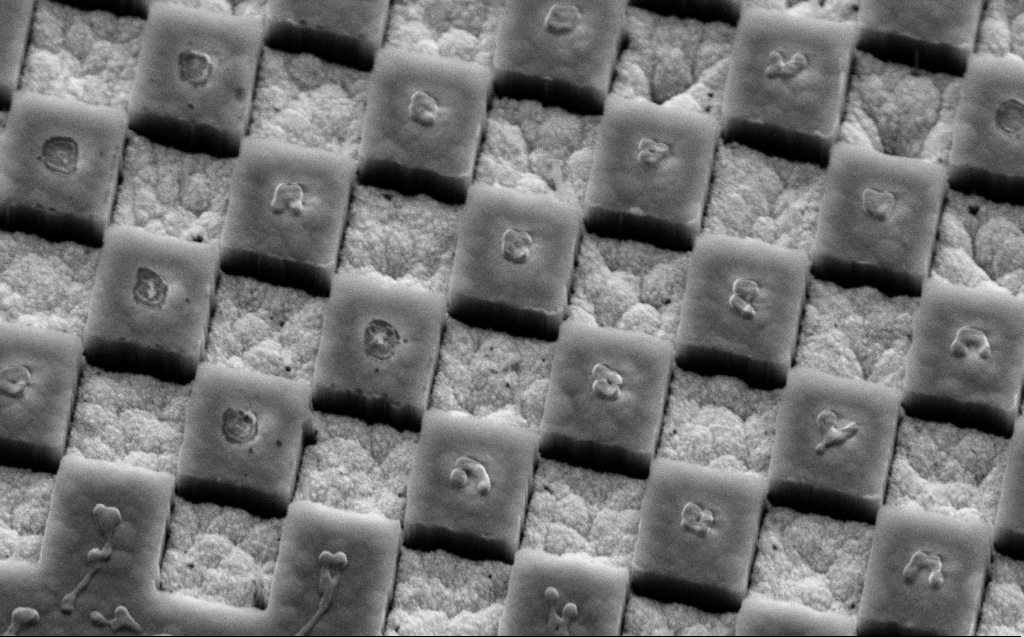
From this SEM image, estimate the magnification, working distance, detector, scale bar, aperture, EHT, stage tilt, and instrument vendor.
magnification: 42.63 K X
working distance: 9 mm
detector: SE2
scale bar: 1000 nm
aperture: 30 µm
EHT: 5 kV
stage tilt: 45°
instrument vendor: Zeiss